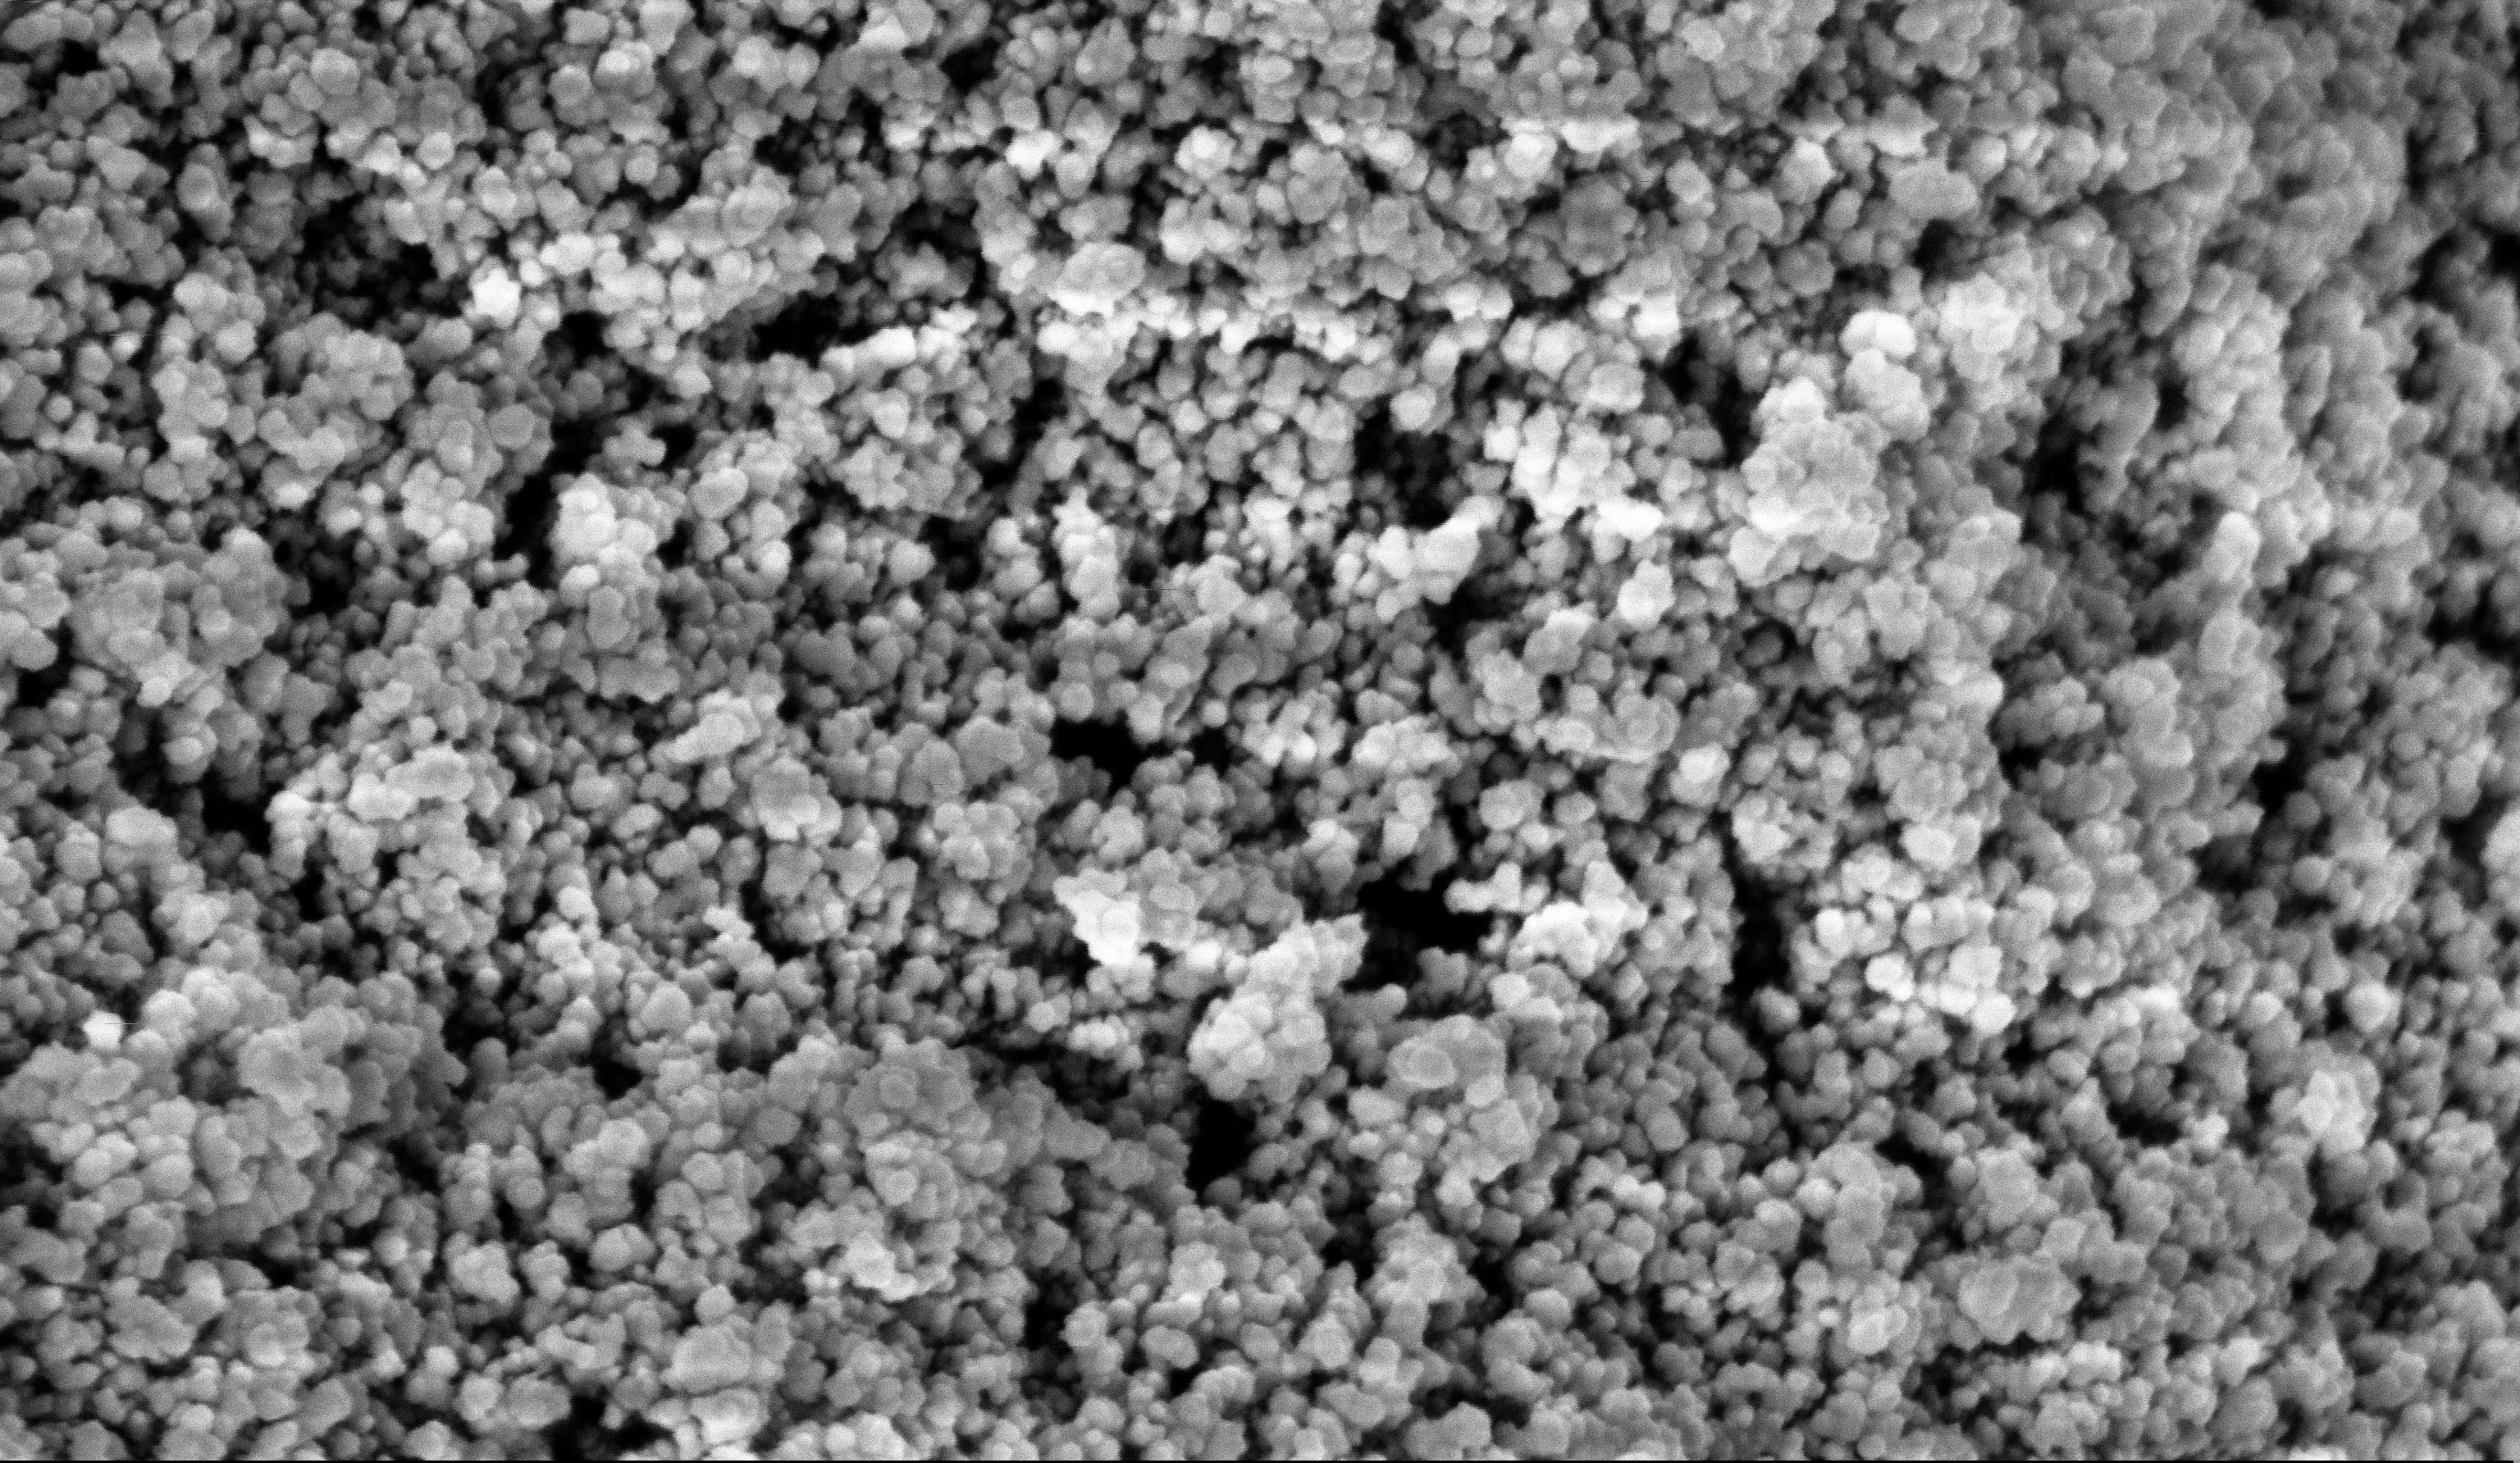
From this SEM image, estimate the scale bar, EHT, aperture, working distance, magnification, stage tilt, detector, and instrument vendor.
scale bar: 100 nm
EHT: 5 kV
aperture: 30 µm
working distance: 5.9 mm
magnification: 135 K X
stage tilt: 0°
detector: InLens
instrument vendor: Zeiss